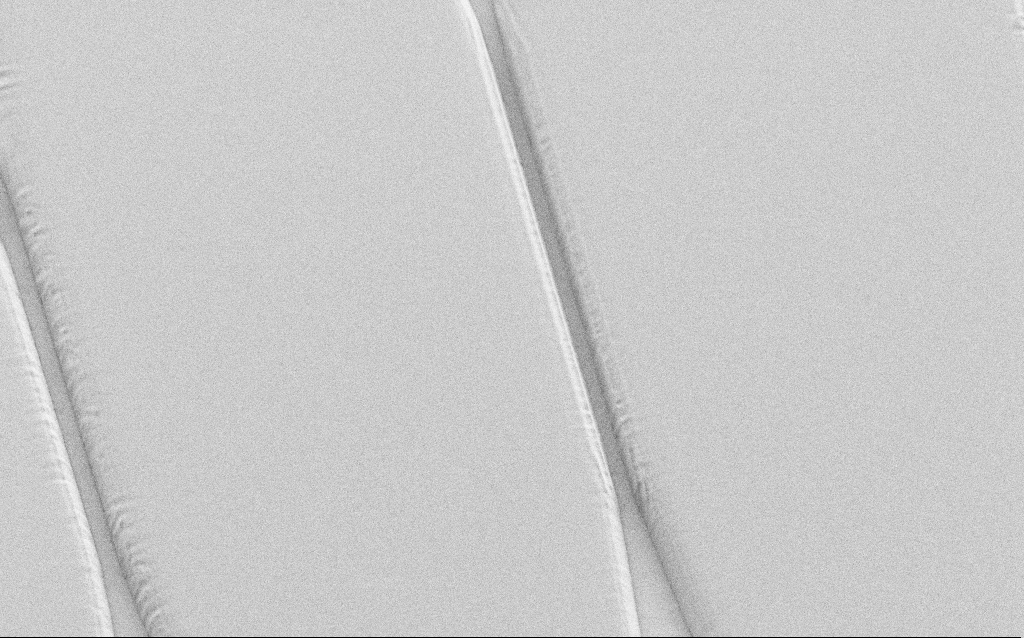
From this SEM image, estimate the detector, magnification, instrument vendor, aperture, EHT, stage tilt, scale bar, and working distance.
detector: SE2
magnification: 1.84 K X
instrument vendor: Zeiss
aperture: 30 µm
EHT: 1 kV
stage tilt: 36°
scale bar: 20000 nm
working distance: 5 mm